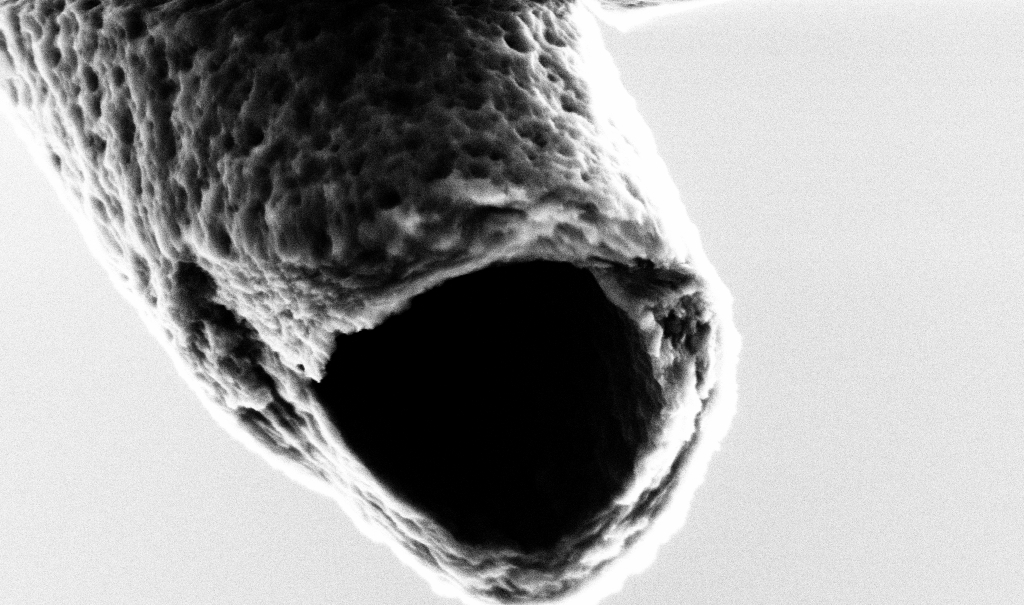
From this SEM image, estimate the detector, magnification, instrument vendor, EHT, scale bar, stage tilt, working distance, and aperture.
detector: SE2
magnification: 75 K X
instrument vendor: Zeiss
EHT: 3 kV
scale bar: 200 nm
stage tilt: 45°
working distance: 7.4 mm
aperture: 30 µm